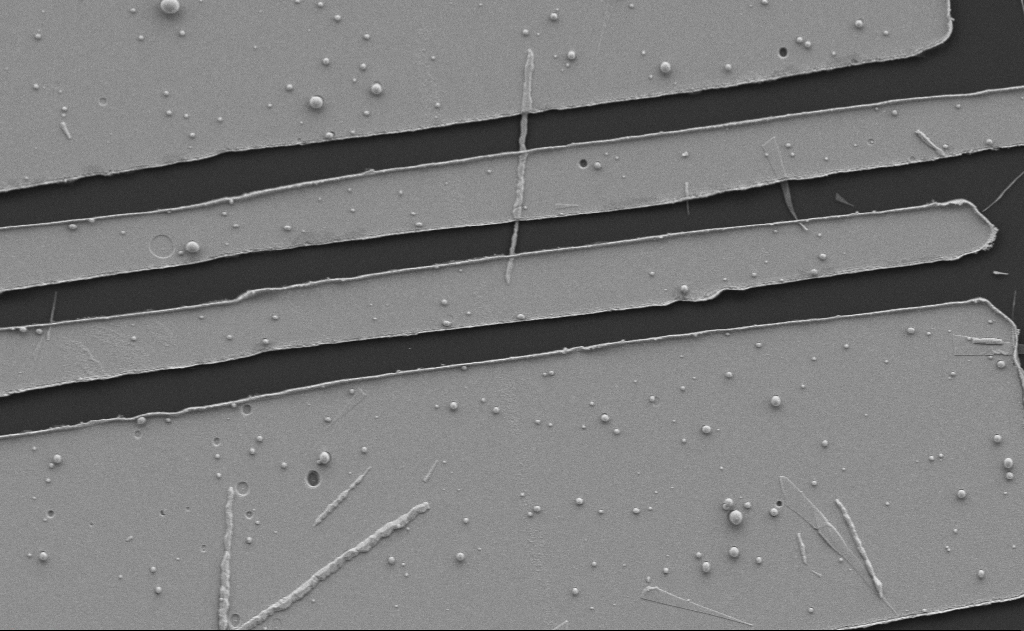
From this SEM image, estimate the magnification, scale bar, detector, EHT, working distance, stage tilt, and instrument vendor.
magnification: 9.71 K X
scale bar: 2000 nm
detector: SE2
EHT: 5 kV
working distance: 10 mm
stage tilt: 0°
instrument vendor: Zeiss